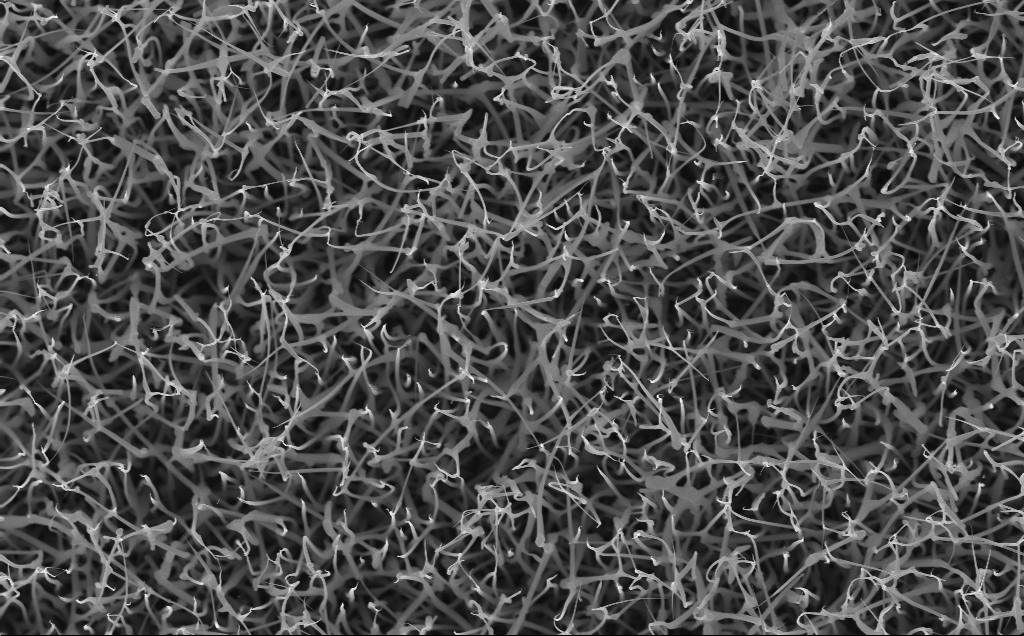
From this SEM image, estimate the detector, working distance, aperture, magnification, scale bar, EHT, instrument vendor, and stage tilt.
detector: InLens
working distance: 5 mm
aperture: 30 µm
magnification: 20 K X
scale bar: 2000 nm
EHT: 10 kV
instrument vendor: Zeiss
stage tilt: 0°